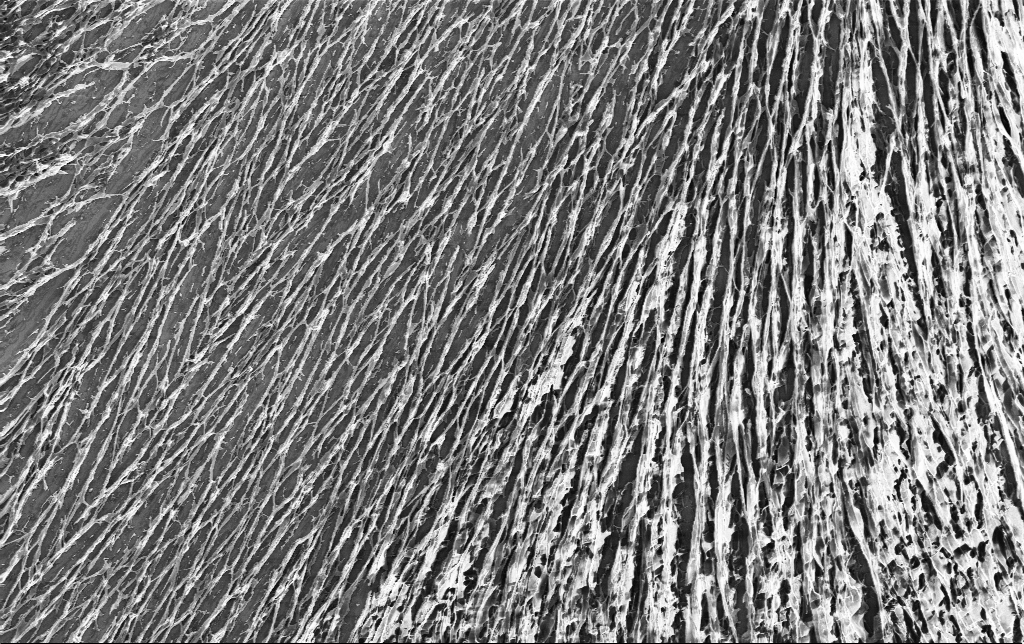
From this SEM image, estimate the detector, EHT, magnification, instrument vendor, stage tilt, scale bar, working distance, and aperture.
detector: InLens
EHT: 3 kV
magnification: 2.31 K X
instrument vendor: Zeiss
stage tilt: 0°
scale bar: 10000 nm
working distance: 3.2 mm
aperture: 30 µm